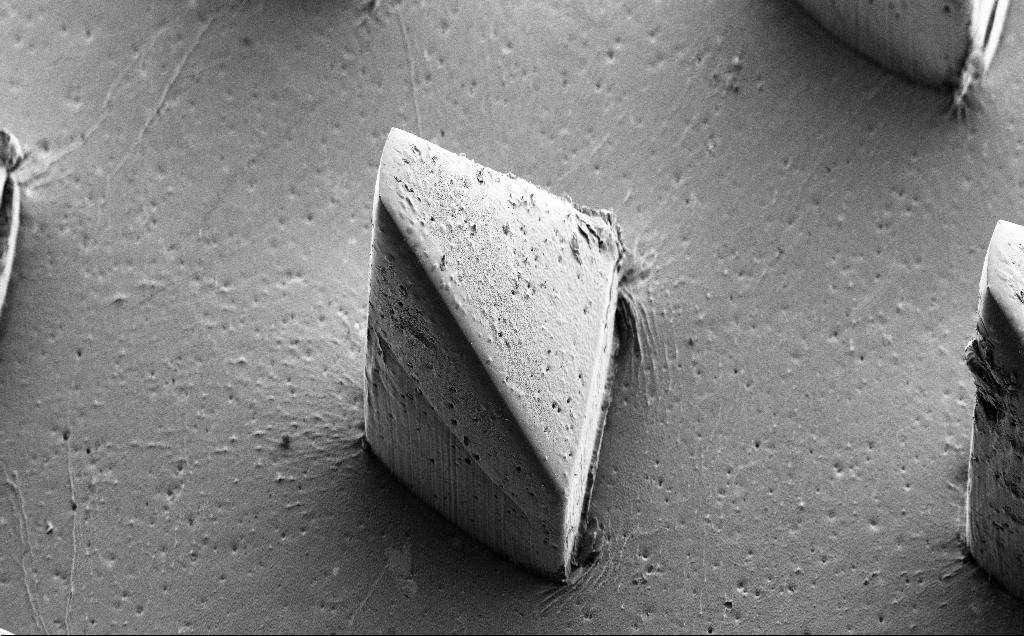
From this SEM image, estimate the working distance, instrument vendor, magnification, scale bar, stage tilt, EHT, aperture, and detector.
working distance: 8 mm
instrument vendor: Zeiss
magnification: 0.243 K X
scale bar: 200000 nm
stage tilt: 39°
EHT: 5 kV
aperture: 30 µm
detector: SE2